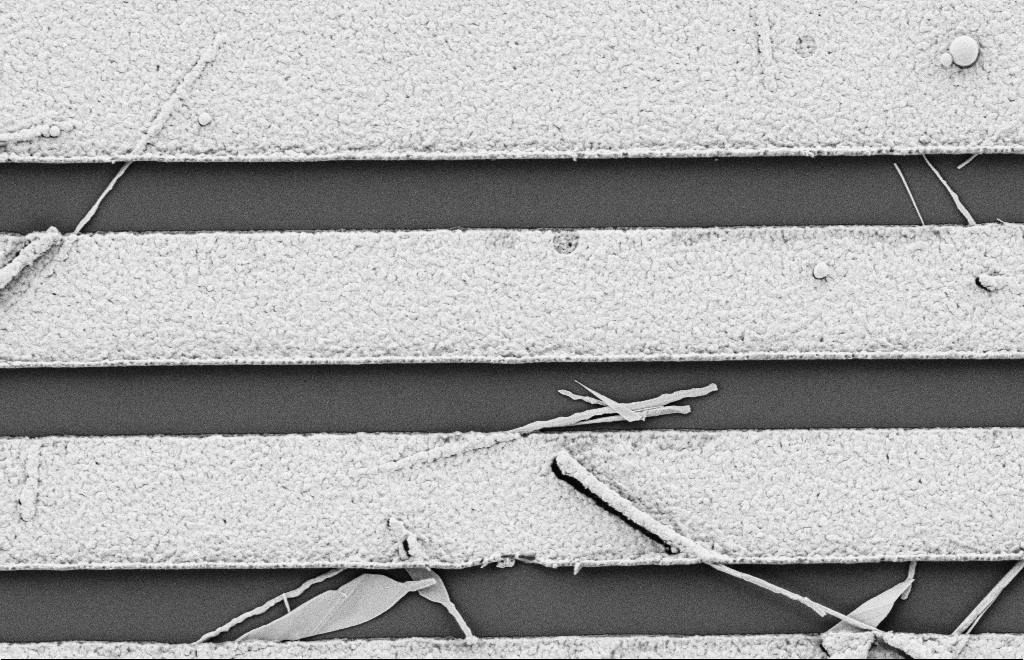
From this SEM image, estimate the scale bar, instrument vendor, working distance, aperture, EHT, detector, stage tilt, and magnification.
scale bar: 2000 nm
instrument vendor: Zeiss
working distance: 10 mm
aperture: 20 µm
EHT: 2 kV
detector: SE2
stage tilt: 0°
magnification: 18.54 K X